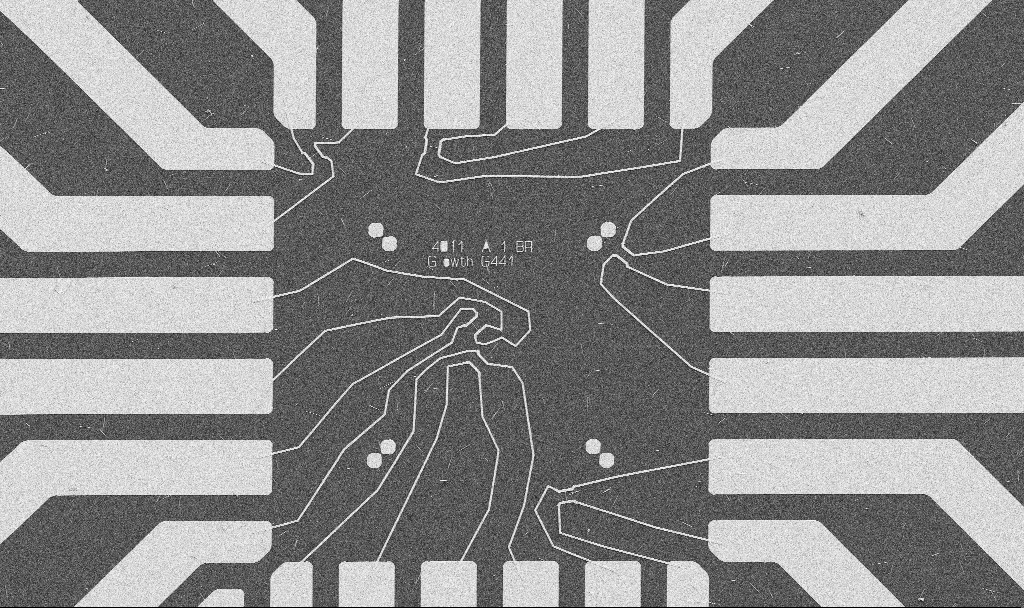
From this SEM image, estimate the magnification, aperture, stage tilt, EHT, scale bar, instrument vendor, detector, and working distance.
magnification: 1 K X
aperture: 30 µm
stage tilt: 0°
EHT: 5 kV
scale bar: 20000 nm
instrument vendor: Zeiss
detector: SE2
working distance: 10.7 mm